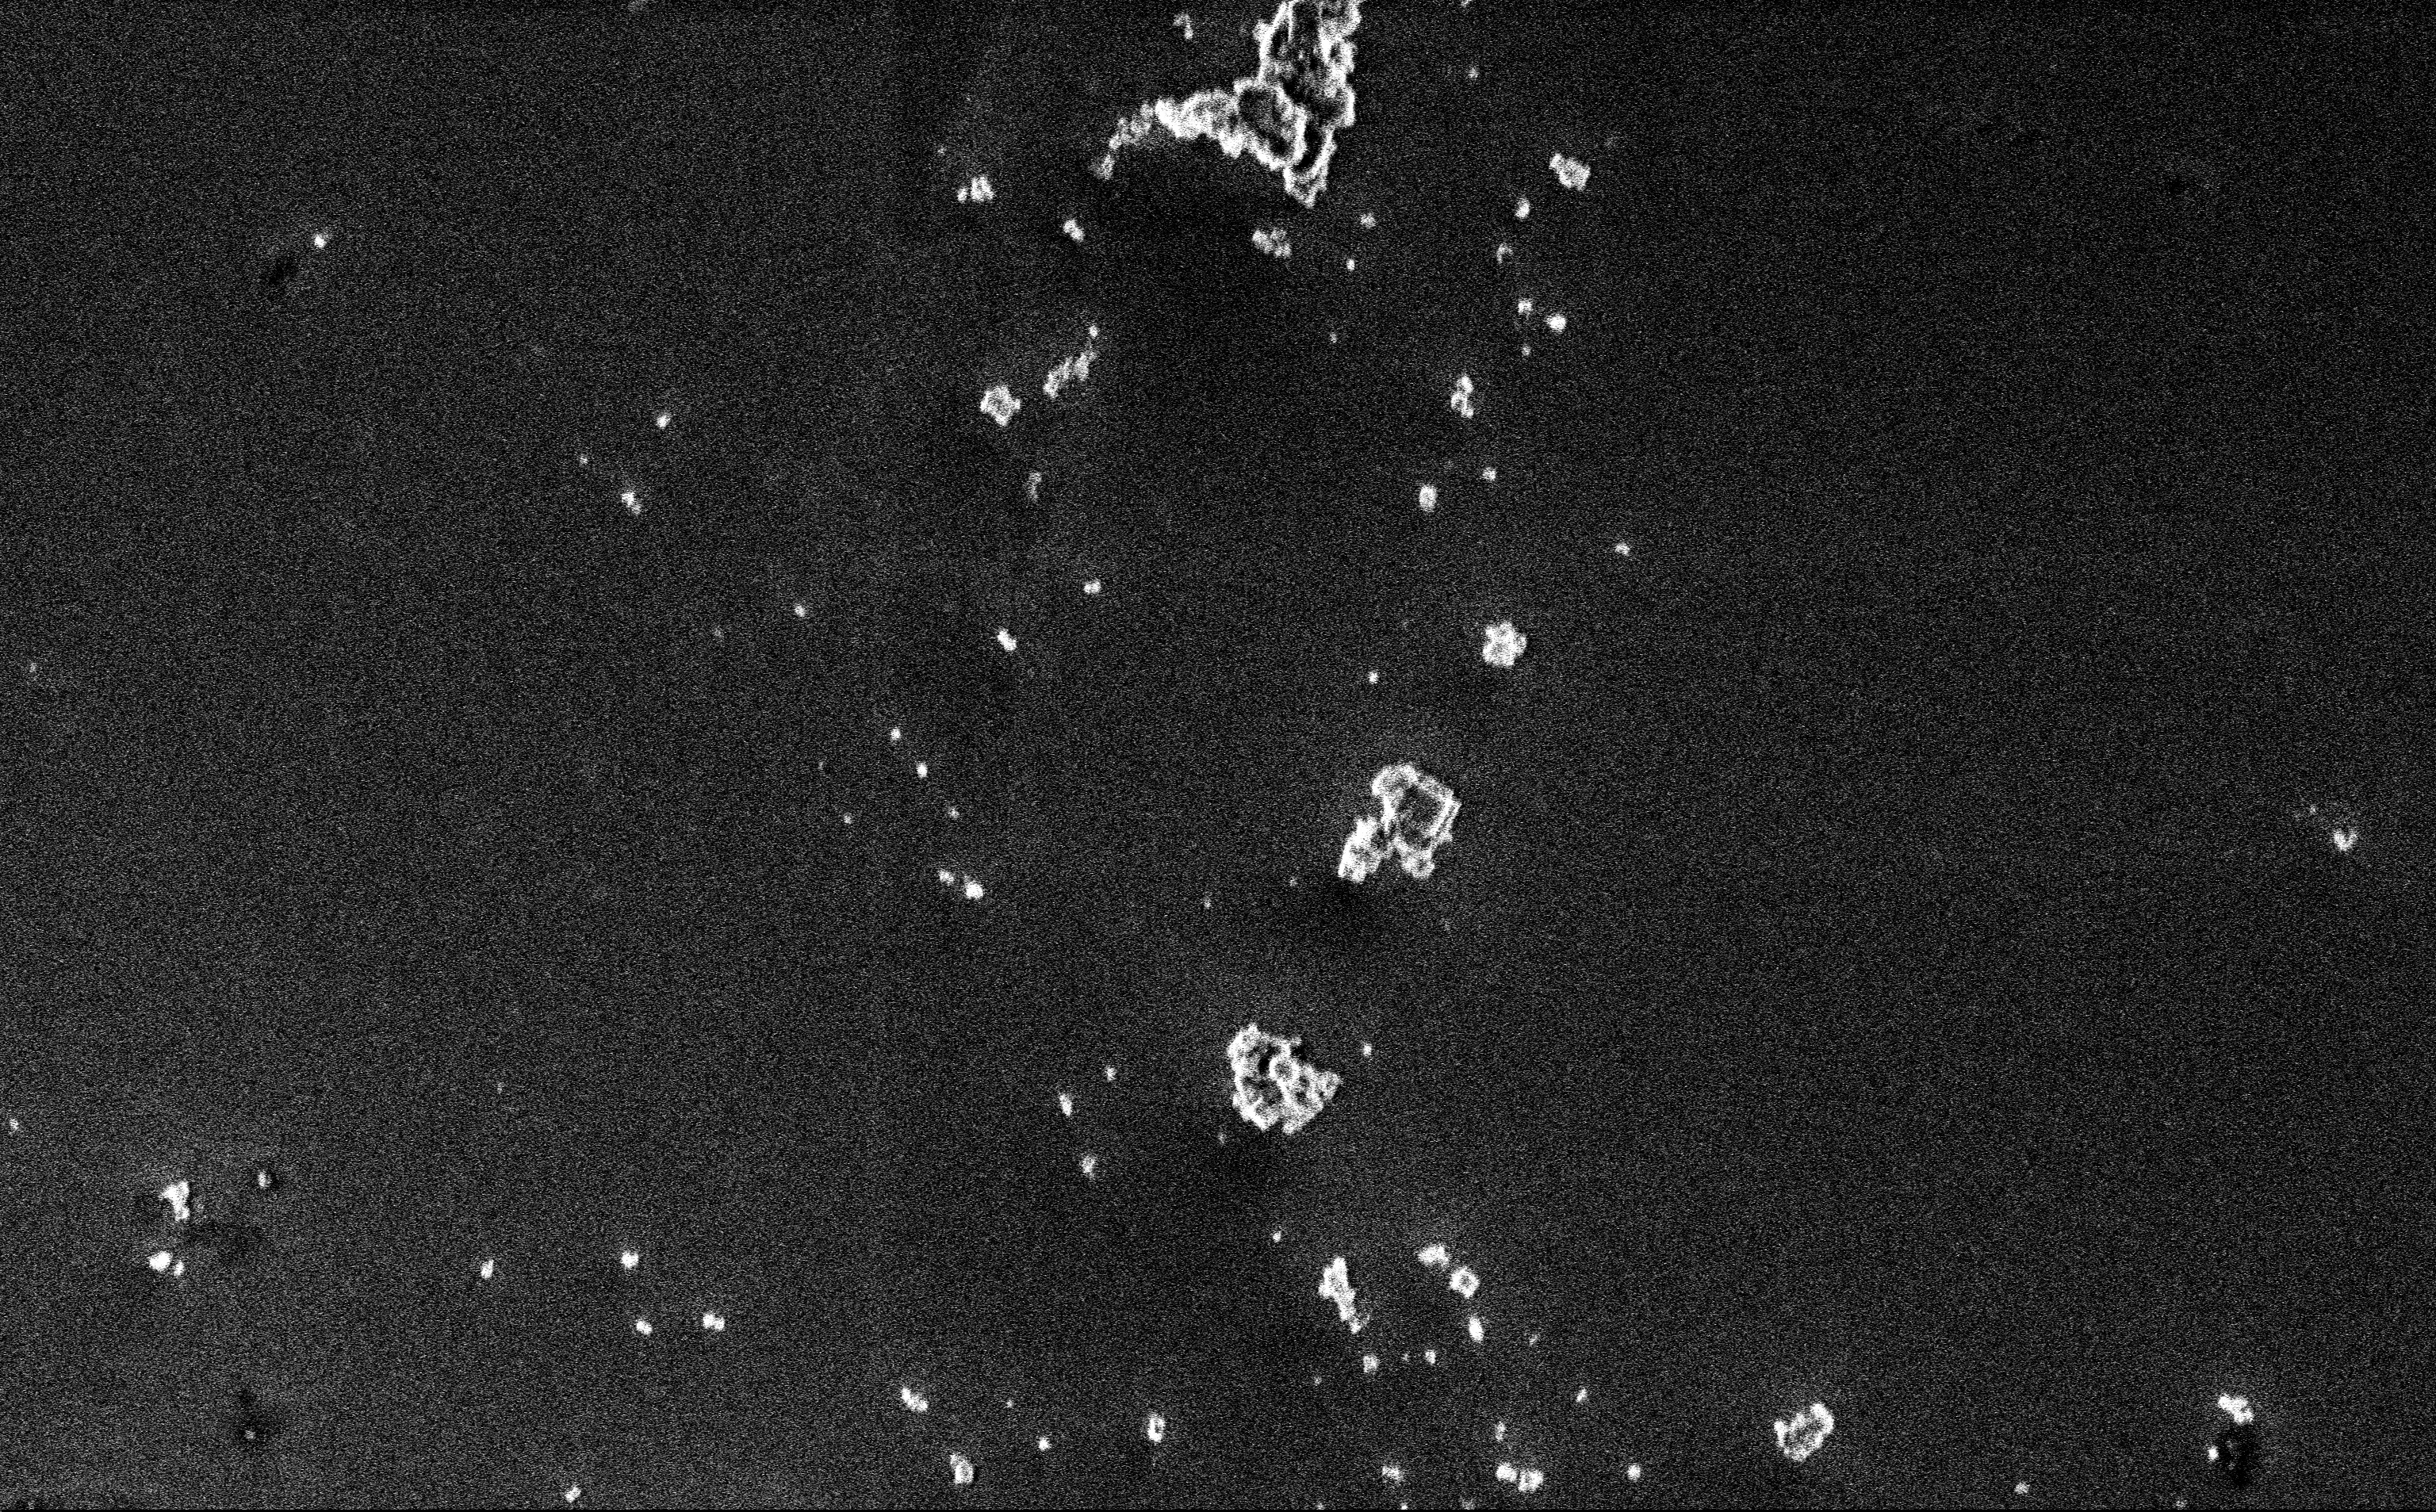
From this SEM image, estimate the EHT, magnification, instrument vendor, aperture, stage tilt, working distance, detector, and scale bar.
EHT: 3 kV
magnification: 12.85 K X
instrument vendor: Zeiss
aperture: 30 µm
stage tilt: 0°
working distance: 3 mm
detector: InLens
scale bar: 2000 nm